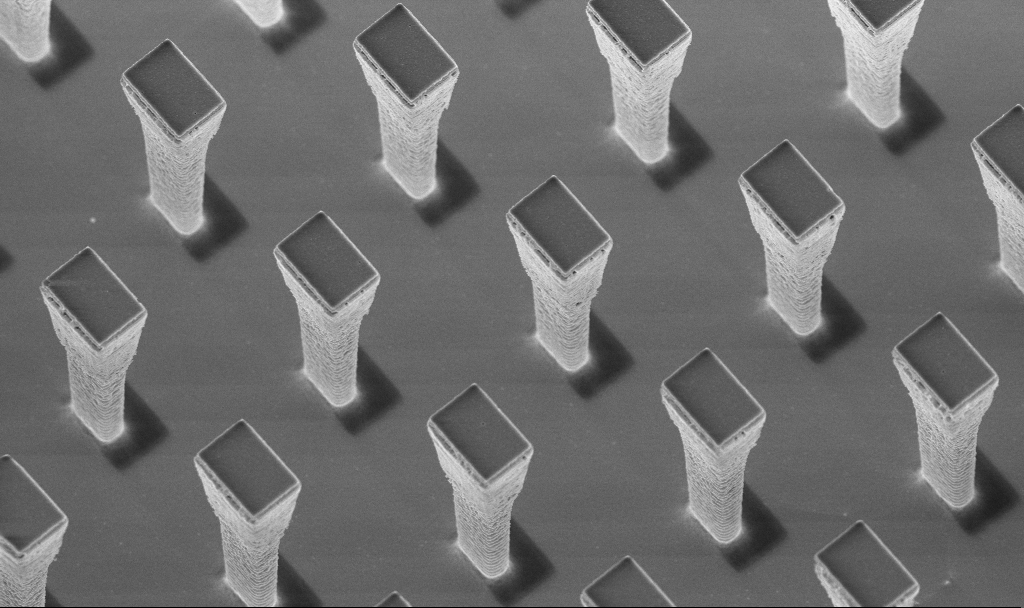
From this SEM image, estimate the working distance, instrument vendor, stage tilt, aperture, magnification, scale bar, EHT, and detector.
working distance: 4.3 mm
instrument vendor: Zeiss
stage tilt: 20°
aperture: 30 µm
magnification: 7.38 K X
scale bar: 2000 nm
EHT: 5 kV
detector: InLens